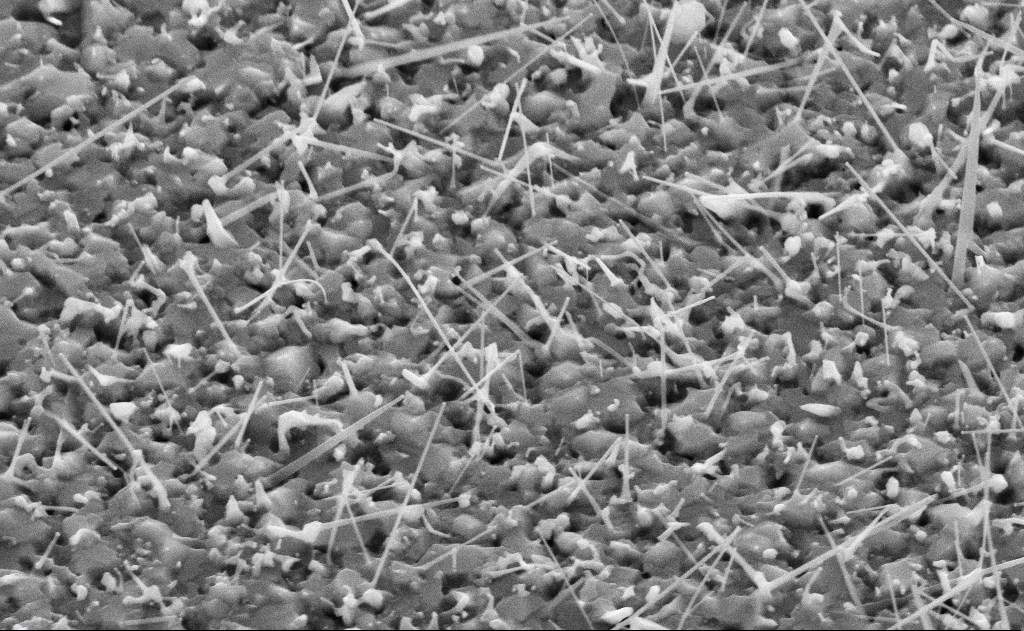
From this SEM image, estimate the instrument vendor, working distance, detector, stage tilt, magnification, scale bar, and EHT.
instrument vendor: Zeiss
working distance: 11 mm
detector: SE2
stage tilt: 45°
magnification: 40 K X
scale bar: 1000 nm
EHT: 10 kV